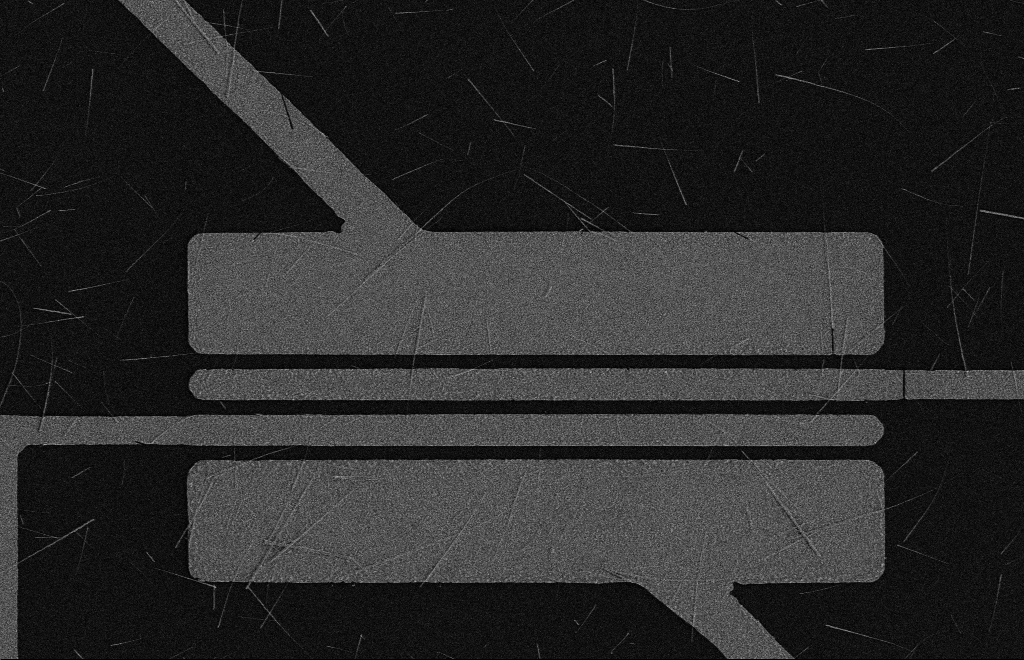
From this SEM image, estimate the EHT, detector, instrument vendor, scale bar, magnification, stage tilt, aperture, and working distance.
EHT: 5 kV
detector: SE2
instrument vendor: Zeiss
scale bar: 10000 nm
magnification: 4.21 K X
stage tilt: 0°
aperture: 10 µm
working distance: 16 mm